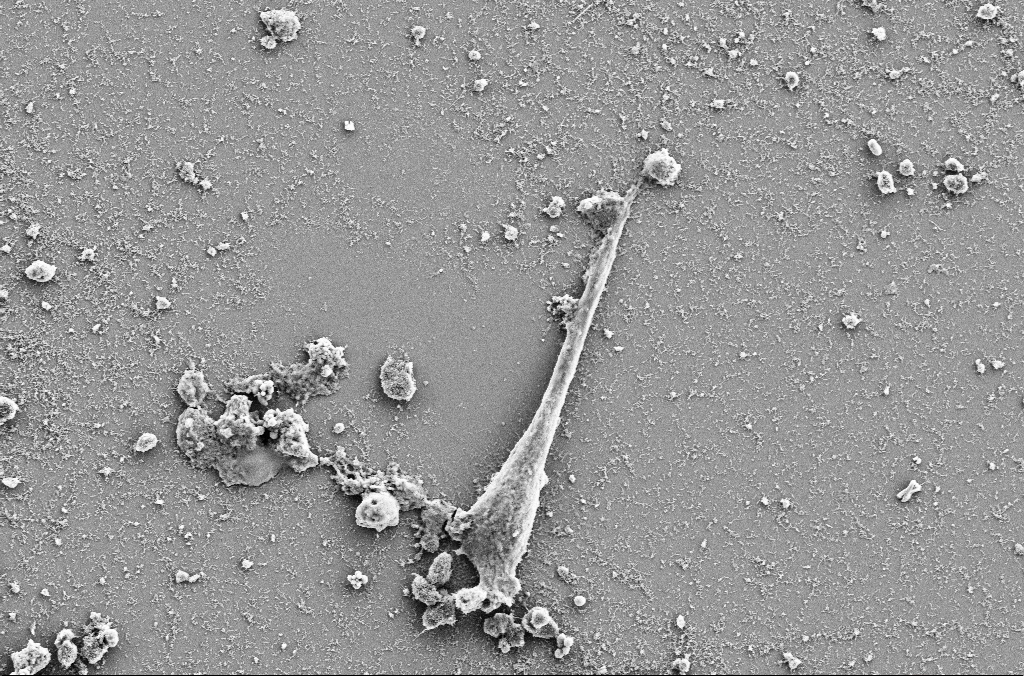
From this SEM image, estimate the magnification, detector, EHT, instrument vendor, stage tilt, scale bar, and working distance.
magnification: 3.5 K X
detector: SE2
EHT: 5 kV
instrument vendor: Zeiss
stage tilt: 0°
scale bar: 10000 nm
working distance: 4 mm